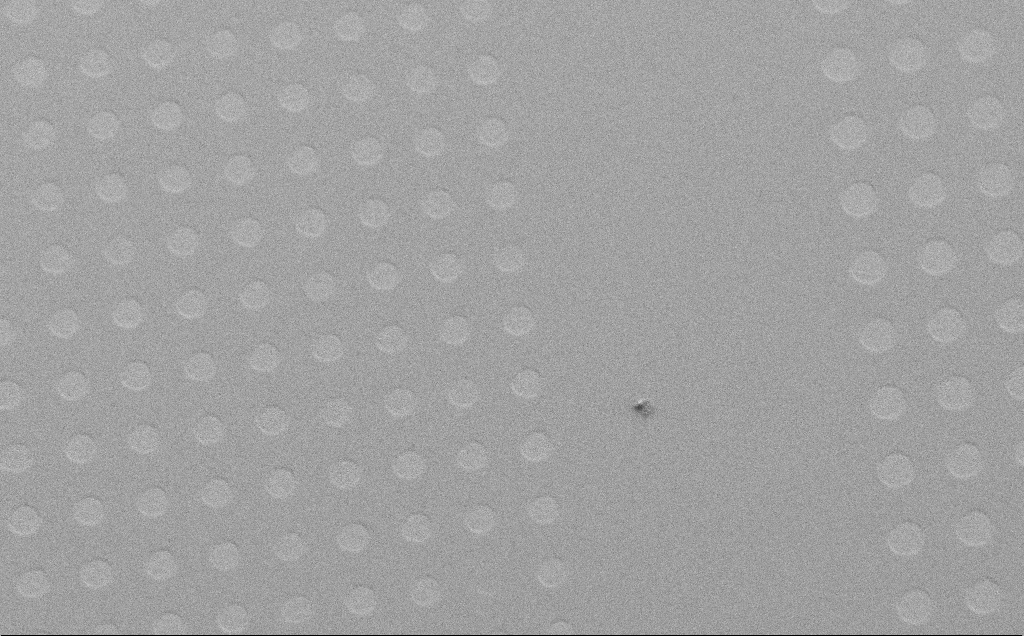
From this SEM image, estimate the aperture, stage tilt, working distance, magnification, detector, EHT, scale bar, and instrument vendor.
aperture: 30 µm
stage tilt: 40.4°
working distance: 6 mm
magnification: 0.916 K X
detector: SE2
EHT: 10 kV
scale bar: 20000 nm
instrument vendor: Zeiss